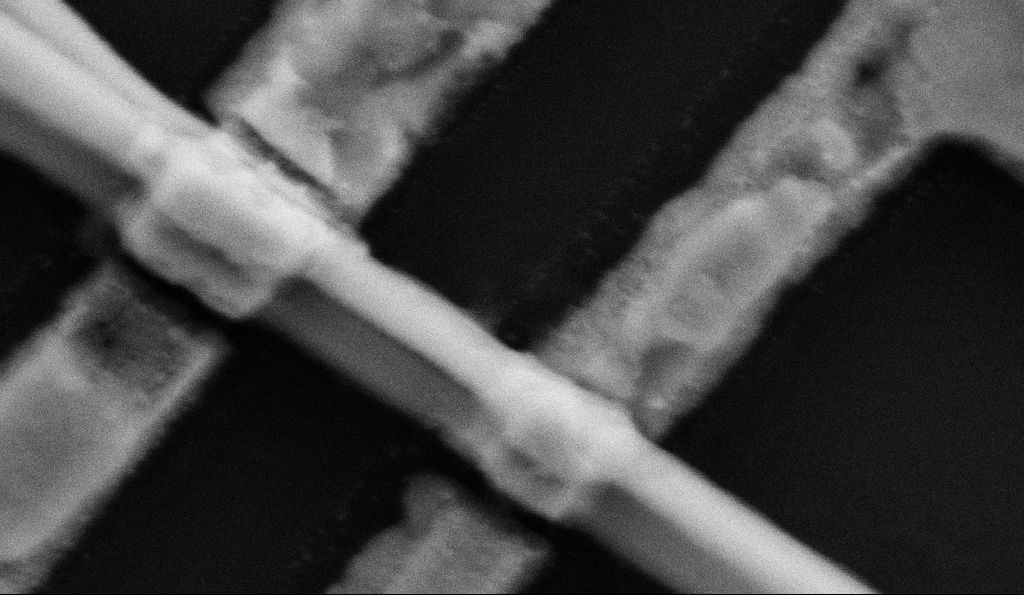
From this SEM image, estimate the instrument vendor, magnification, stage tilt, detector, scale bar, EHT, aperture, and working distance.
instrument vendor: Zeiss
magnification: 228.15 K X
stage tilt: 0°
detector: SE2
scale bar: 200 nm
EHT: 5 kV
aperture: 30 µm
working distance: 8.5 mm